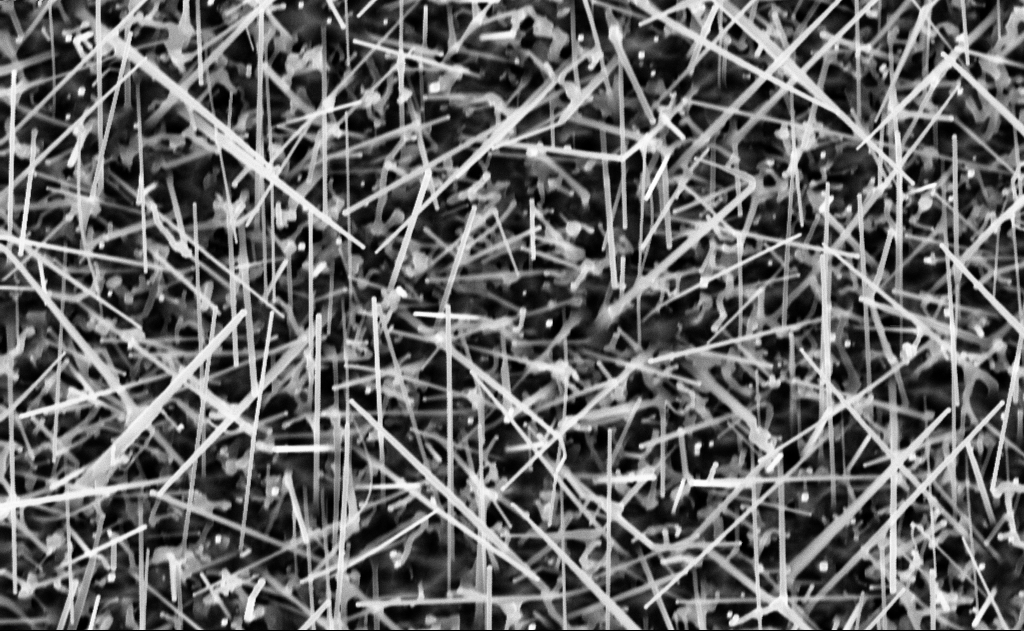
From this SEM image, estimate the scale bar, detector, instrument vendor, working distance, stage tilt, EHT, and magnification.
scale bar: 1000 nm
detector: InLens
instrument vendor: Zeiss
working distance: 10 mm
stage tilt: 0°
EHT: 10 kV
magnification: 40 K X